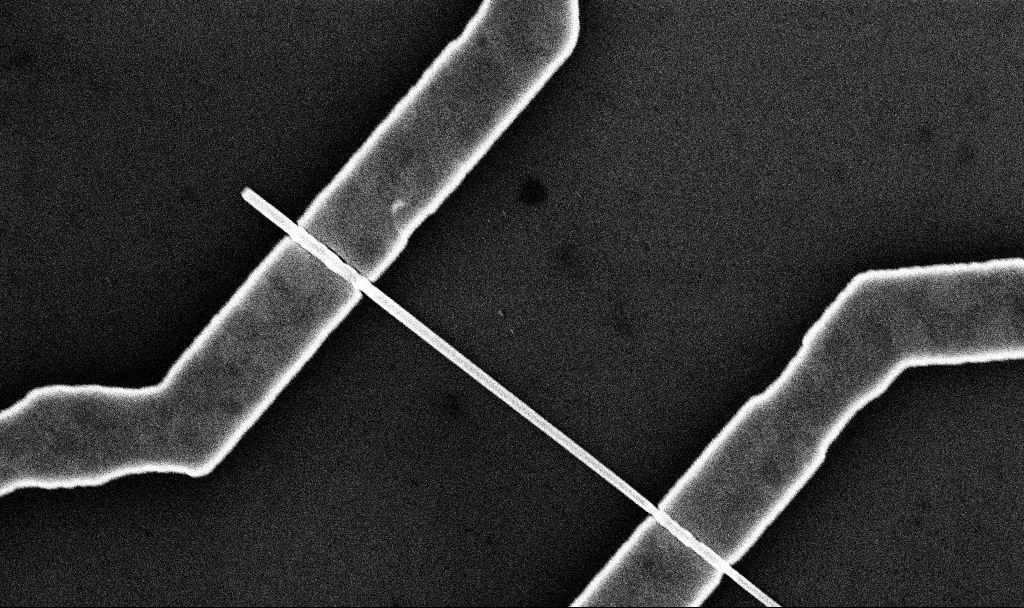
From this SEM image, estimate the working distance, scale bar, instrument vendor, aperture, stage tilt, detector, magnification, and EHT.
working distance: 6.7 mm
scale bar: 1000 nm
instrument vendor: Zeiss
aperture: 30 µm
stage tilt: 0°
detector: InLens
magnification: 39.21 K X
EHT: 10 kV